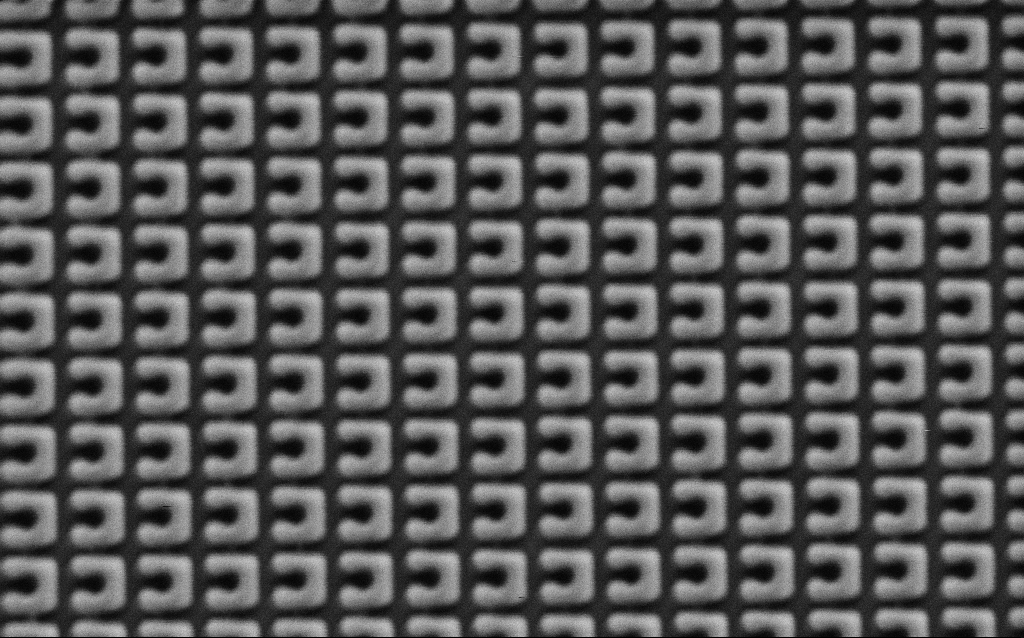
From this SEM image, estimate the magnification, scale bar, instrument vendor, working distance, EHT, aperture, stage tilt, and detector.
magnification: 52.5 K X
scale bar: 1000 nm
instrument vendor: Zeiss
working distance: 9.8 mm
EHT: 1.5 kV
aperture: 30 µm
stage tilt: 0°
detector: SE2